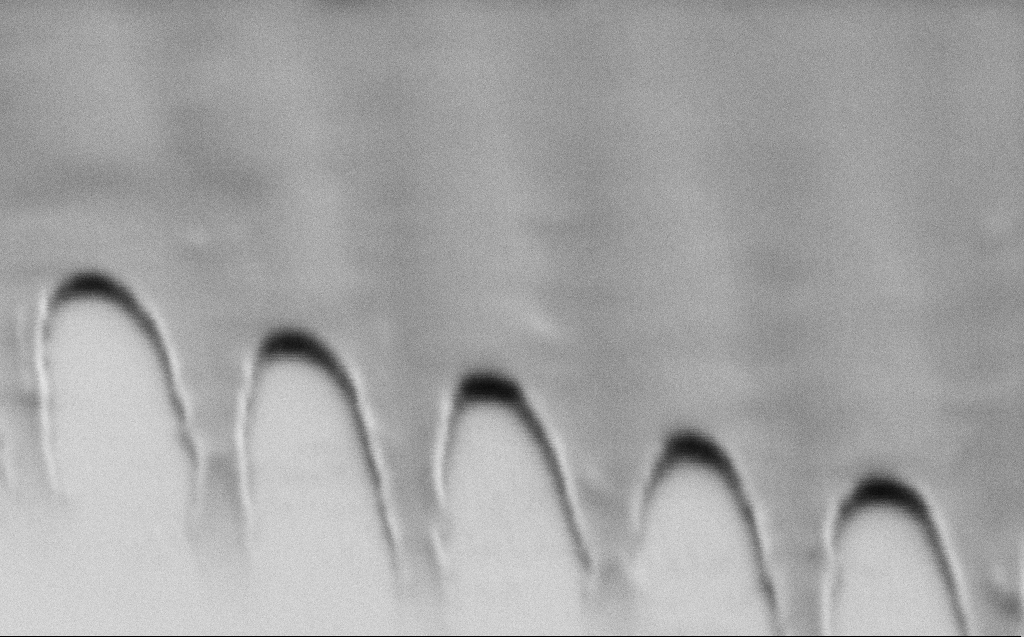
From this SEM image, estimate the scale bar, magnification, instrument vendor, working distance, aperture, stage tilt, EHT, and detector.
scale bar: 200 nm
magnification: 139.92 K X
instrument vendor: Zeiss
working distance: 3 mm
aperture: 30 µm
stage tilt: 60.7°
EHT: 1 kV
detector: SE2